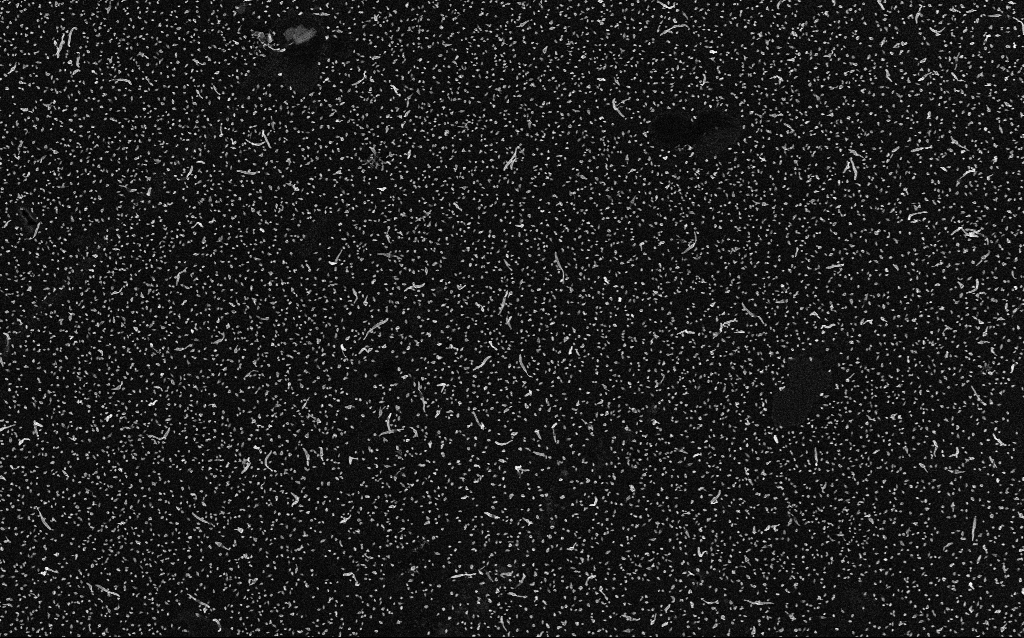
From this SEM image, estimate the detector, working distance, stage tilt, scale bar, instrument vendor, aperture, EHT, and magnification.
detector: InLens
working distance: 2.1 mm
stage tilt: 0°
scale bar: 2000 nm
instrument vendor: Zeiss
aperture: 30 µm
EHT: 5 kV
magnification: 10 K X